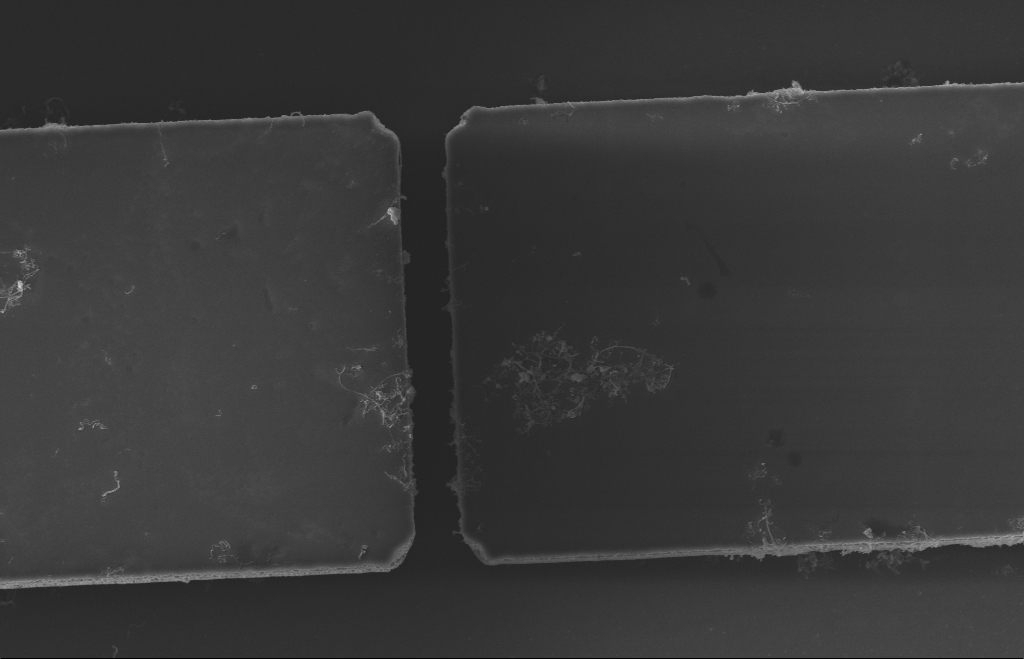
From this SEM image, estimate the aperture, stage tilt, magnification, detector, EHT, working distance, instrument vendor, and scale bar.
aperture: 20 µm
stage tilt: -0.3°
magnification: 8.69 K X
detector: InLens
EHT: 5 kV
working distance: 10 mm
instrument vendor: Zeiss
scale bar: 2000 nm